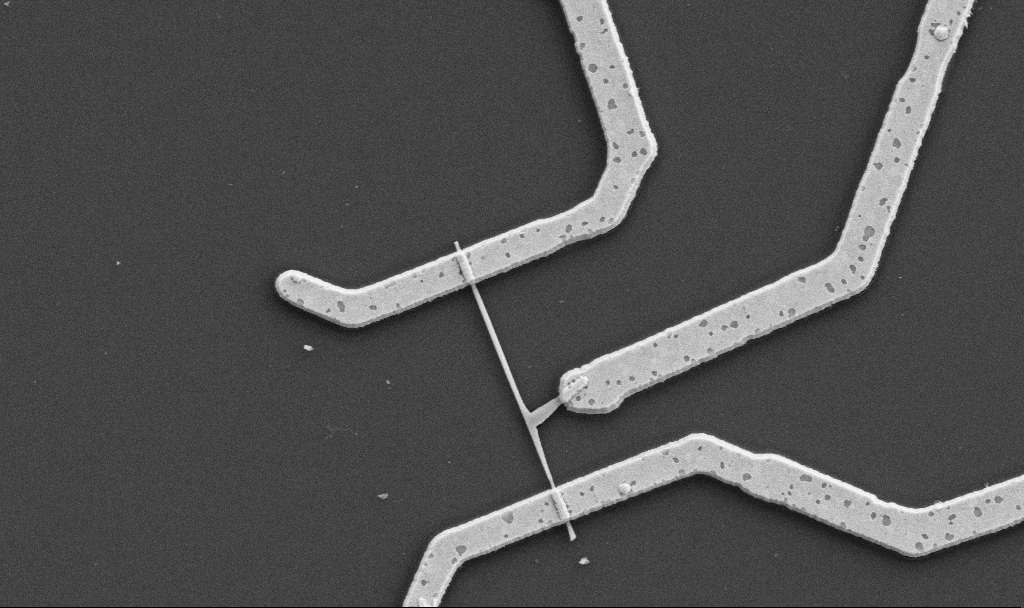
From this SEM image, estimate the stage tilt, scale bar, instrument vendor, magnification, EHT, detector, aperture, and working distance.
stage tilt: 0°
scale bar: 1000 nm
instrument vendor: Zeiss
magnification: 20 K X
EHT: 5 kV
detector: SE2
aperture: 30 µm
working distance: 10.7 mm